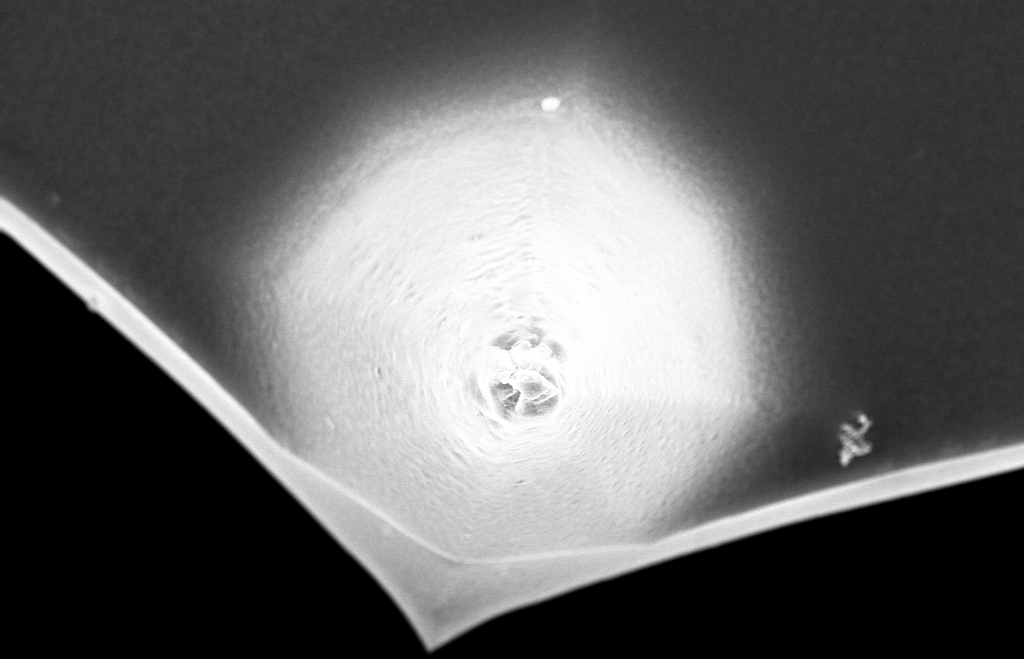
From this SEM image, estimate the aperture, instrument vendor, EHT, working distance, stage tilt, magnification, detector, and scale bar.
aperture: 30 µm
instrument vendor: Zeiss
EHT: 10 kV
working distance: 8 mm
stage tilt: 0°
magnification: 15.45 K X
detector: InLens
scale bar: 2000 nm